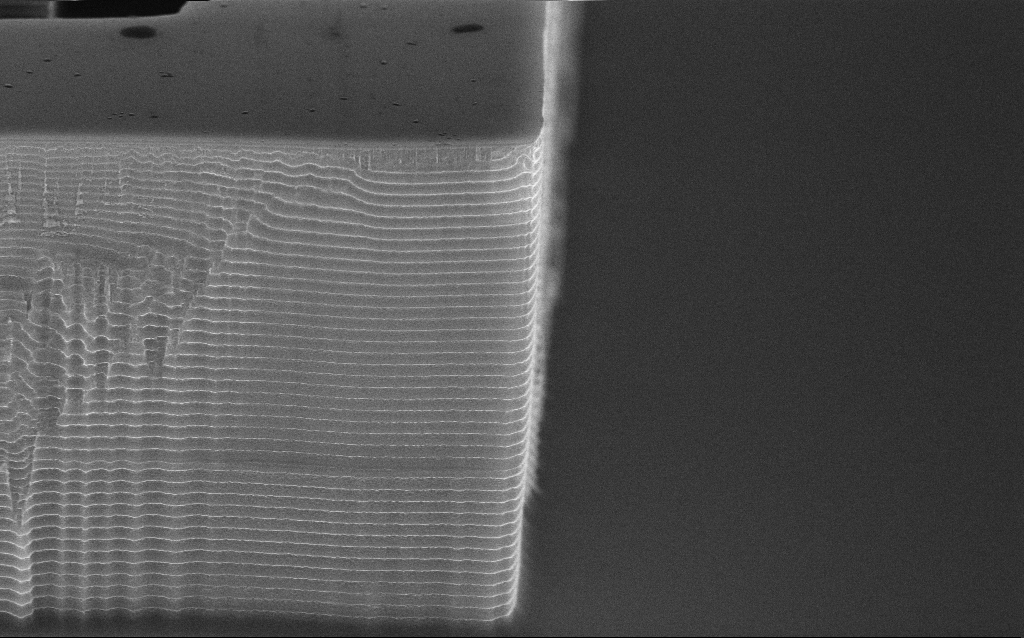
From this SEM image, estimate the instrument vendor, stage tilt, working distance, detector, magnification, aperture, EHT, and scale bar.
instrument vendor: Zeiss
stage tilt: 70°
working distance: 4.4 mm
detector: InLens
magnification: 15 K X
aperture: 30 µm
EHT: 10 kV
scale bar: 2000 nm